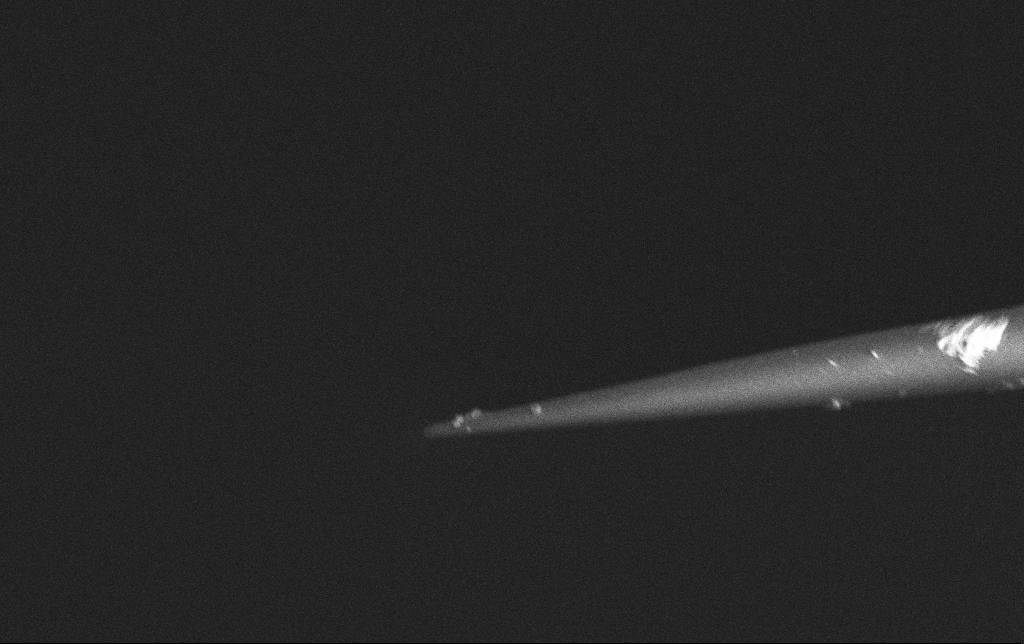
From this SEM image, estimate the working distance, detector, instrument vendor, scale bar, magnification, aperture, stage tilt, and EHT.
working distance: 6.6 mm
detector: InLens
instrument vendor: Zeiss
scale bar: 1000 nm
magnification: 25 K X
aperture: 30 µm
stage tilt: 0°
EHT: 2 kV